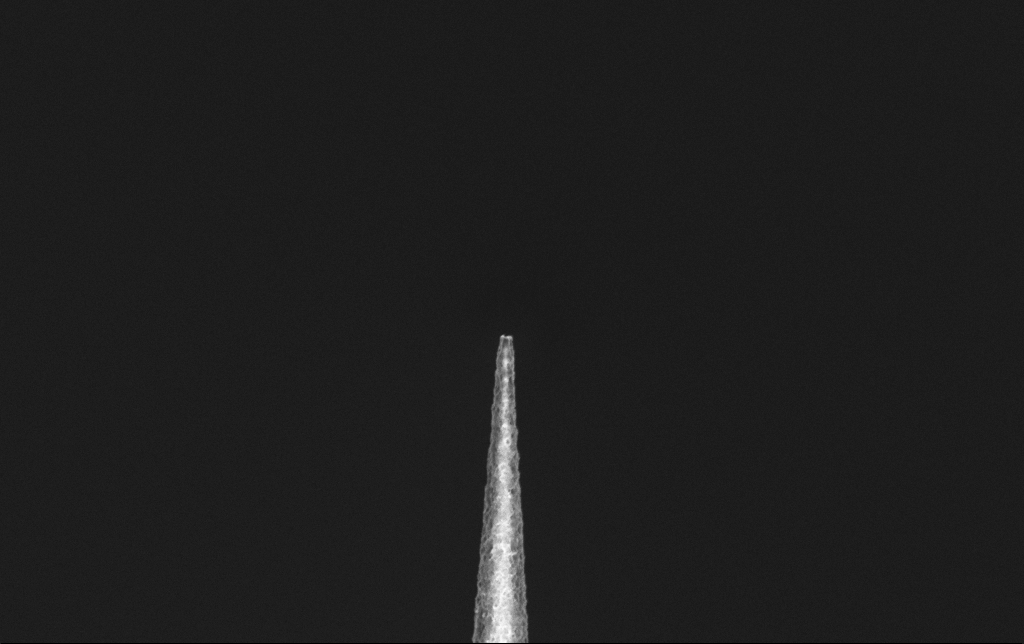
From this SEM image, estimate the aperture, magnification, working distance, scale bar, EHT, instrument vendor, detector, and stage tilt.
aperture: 30 µm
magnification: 25 K X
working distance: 6.5 mm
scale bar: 2000 nm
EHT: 2 kV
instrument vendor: Zeiss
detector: InLens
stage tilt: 0°